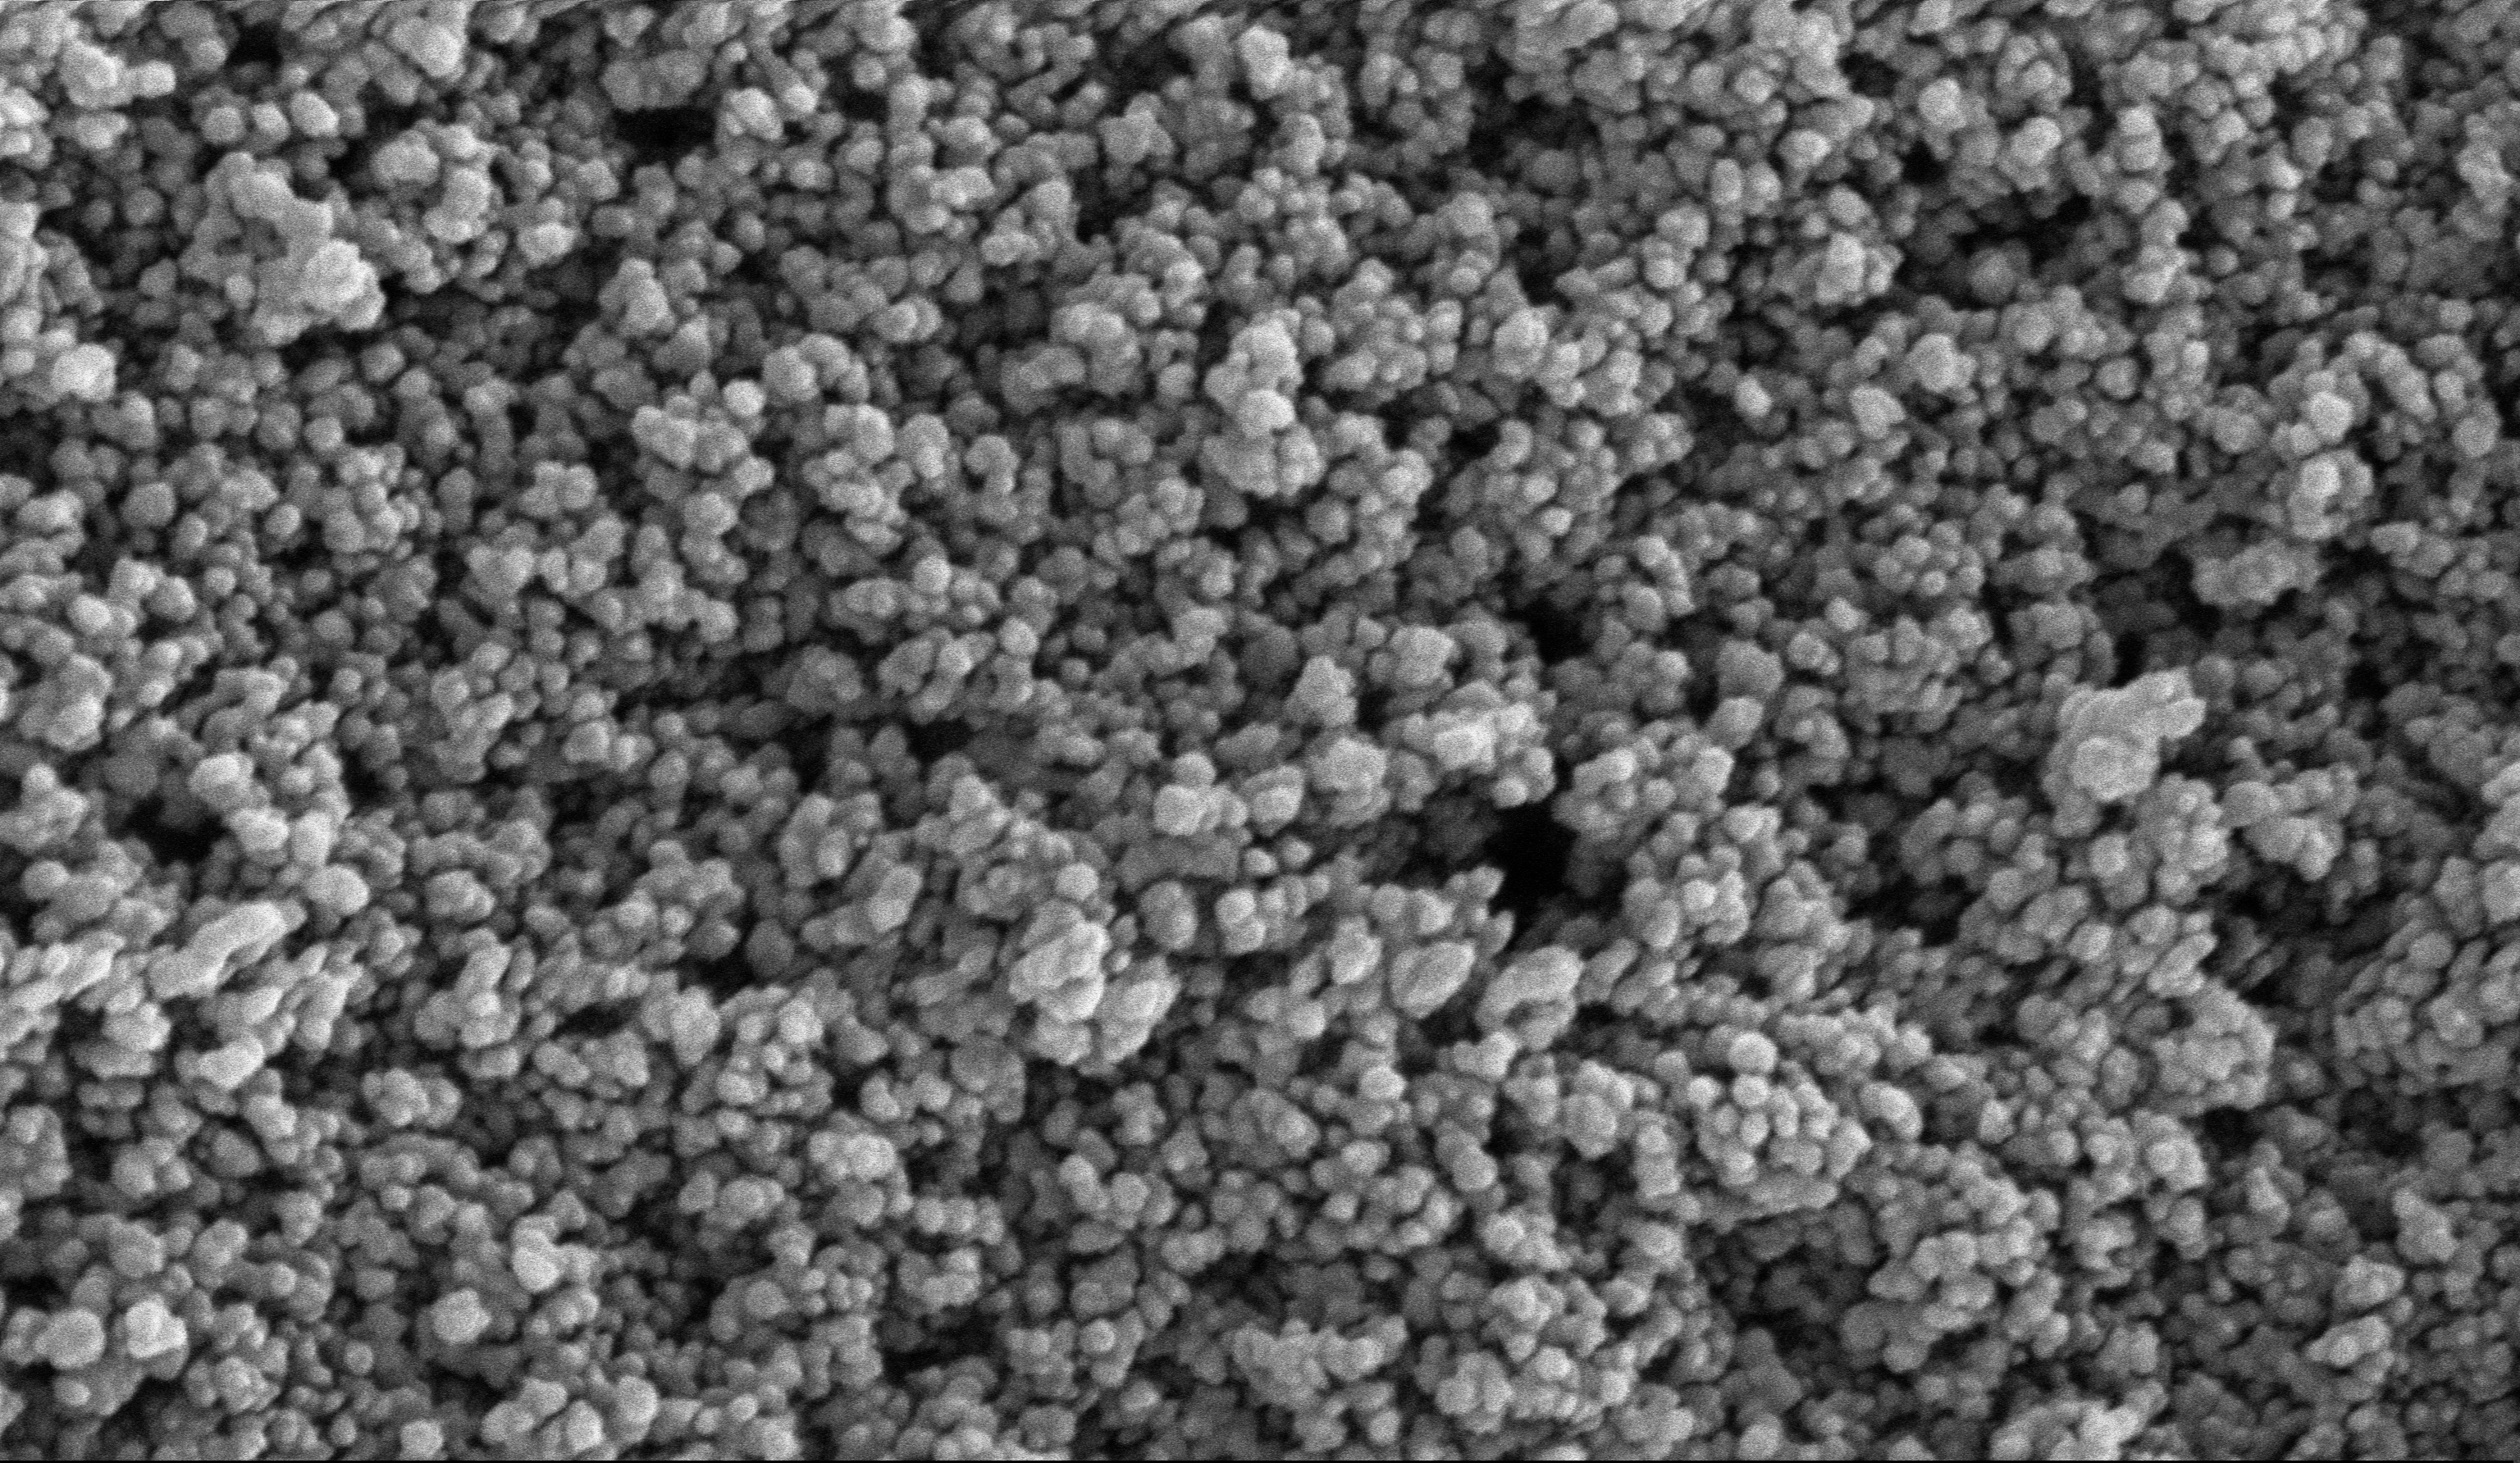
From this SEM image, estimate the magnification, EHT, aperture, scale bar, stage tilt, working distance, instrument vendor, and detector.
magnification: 135 K X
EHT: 10 kV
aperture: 30 µm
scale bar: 100 nm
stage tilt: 0°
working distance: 5.1 mm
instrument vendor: Zeiss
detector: InLens